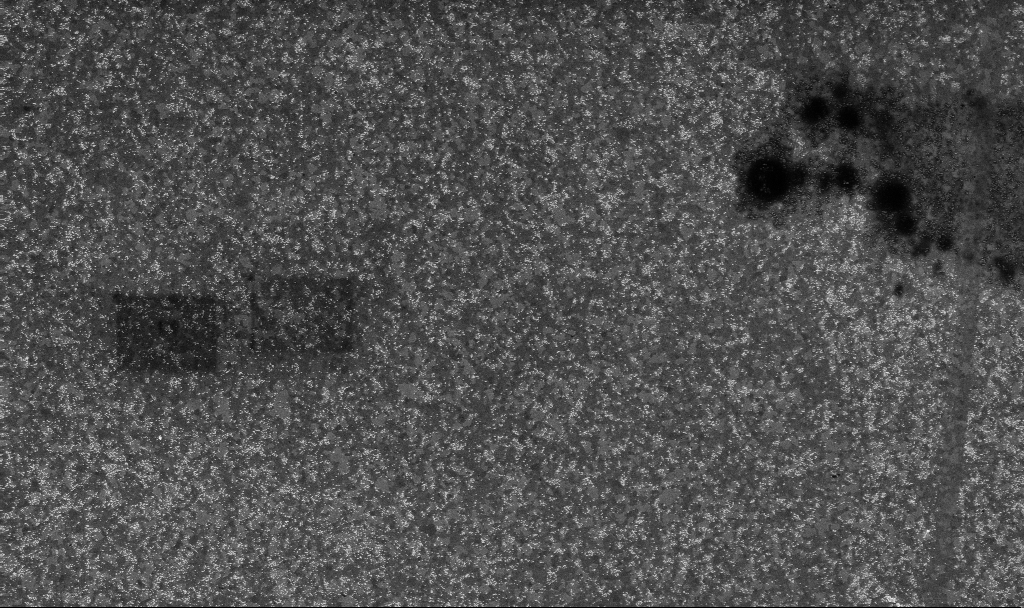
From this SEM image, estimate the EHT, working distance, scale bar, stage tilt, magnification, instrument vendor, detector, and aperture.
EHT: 10 kV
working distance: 3.8 mm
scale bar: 2000 nm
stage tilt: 0°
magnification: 10 K X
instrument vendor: Zeiss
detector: InLens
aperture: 30 µm